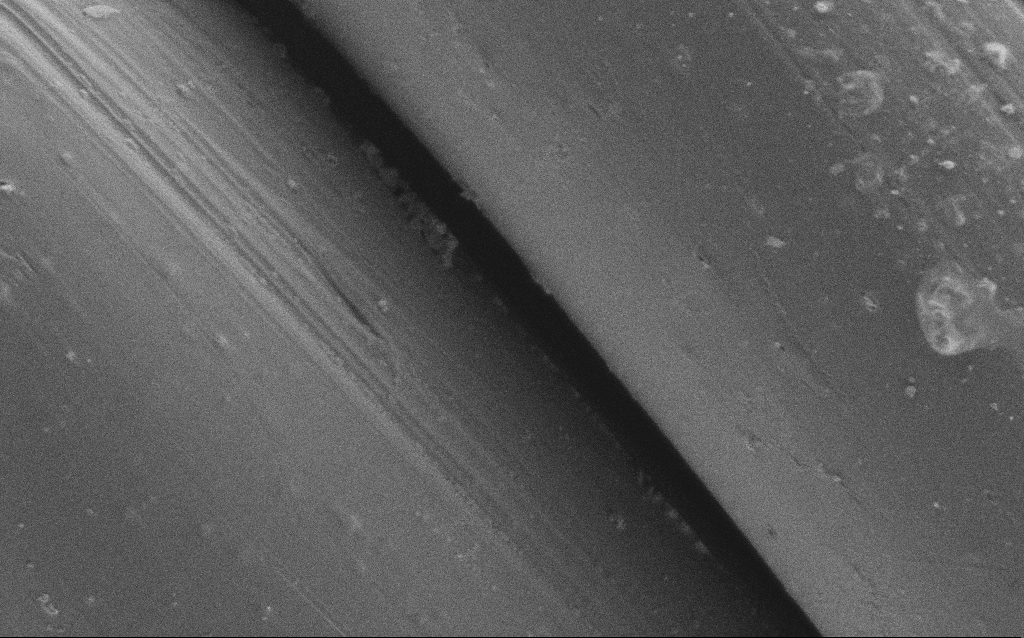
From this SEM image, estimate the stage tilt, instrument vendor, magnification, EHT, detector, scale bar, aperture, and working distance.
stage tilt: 0°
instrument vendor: Zeiss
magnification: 11.58 K X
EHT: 1 kV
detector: SE2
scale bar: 2000 nm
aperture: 30 µm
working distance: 4 mm